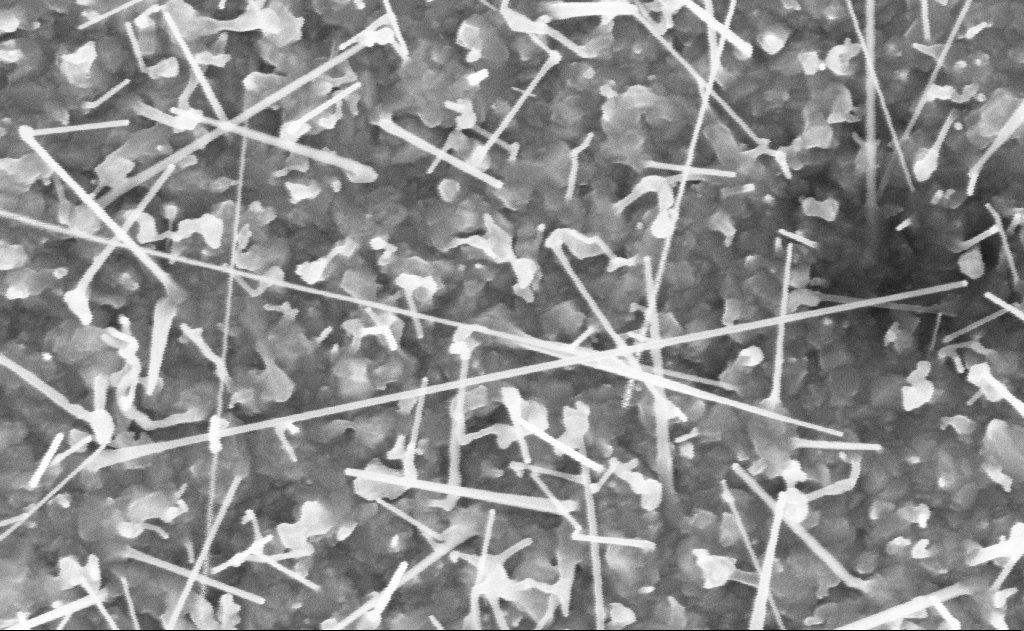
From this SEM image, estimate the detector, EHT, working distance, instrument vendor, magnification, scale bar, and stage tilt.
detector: InLens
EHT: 10 kV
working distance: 20 mm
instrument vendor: Zeiss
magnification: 80 K X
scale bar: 200 nm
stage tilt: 0°